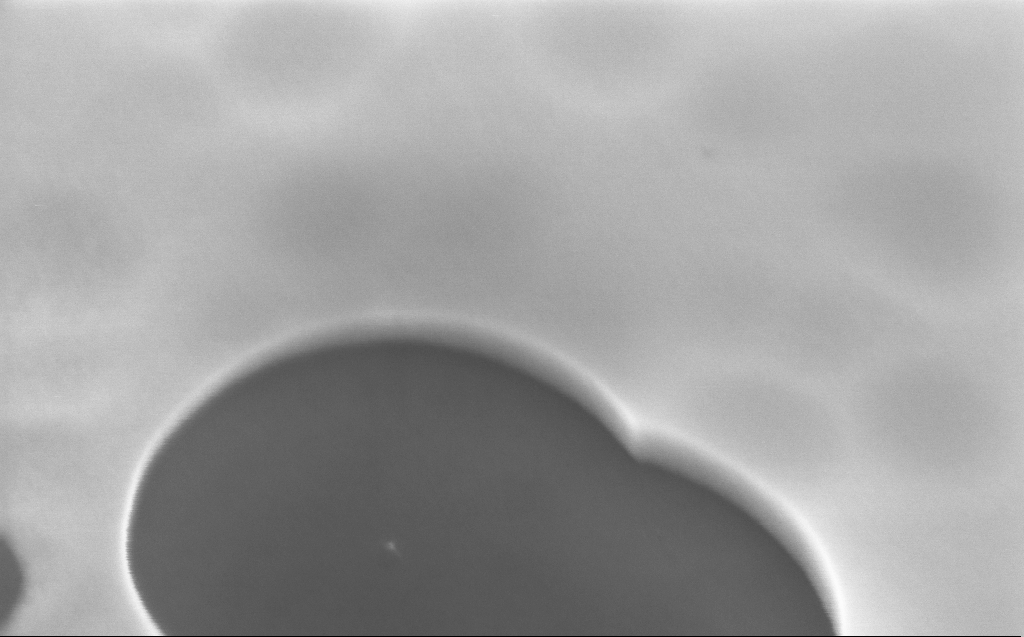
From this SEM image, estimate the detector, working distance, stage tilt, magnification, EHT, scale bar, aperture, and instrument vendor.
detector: InLens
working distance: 4 mm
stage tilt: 45°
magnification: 227.71 K X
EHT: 5 kV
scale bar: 200 nm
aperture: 30 µm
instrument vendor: Zeiss